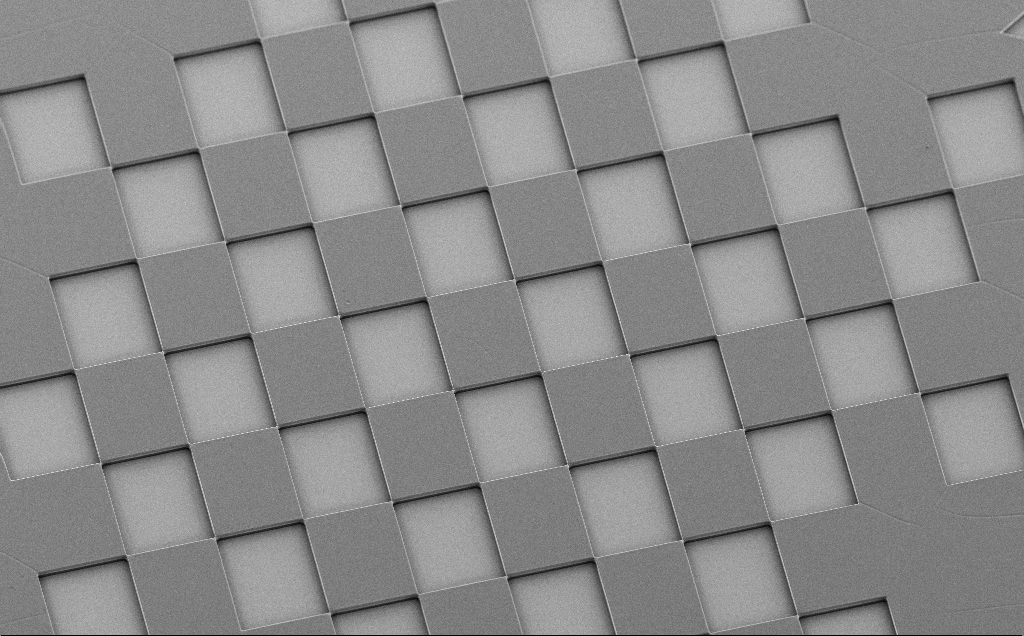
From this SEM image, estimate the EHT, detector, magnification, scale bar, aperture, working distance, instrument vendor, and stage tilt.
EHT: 5 kV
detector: SE2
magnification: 0.35 K X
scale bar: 100000 nm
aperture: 30 µm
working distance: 11 mm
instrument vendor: Zeiss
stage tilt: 30°